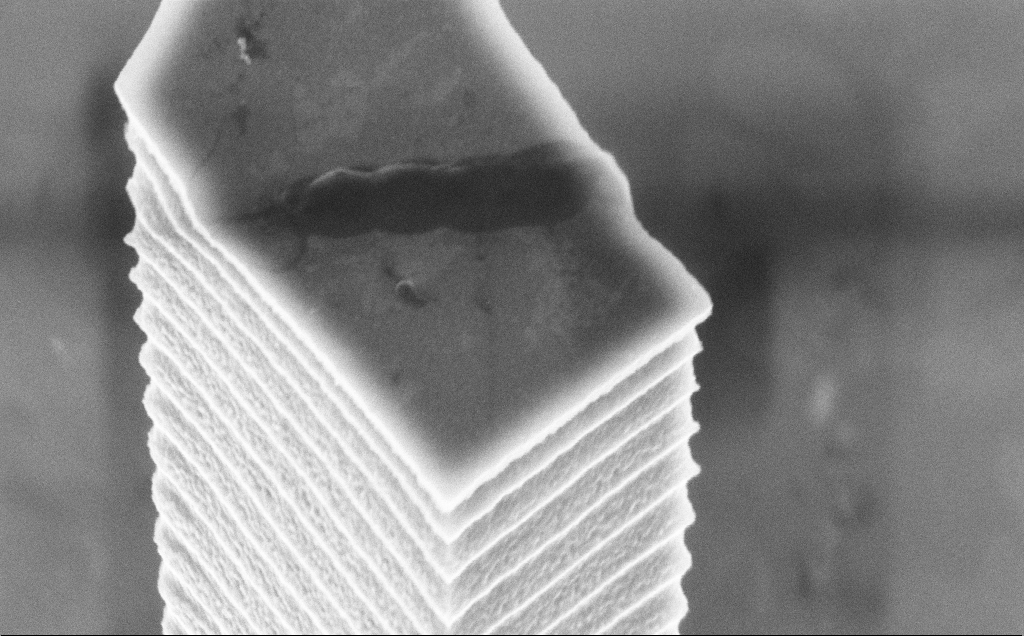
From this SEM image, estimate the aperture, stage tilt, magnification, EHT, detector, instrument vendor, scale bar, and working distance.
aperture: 30 µm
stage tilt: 45°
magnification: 44.15 K X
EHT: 5 kV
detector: InLens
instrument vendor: Zeiss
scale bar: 1000 nm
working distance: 14 mm